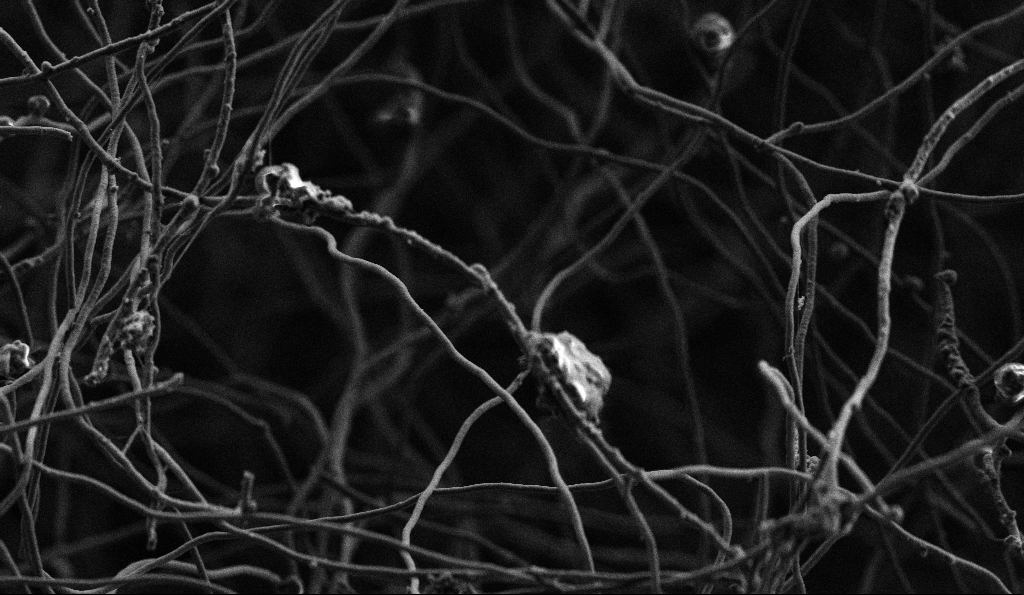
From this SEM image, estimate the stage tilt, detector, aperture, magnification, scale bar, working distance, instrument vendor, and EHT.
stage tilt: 0°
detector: SE2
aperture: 30 µm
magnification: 5 K X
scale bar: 10000 nm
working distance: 5.2 mm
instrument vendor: Zeiss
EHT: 3 kV